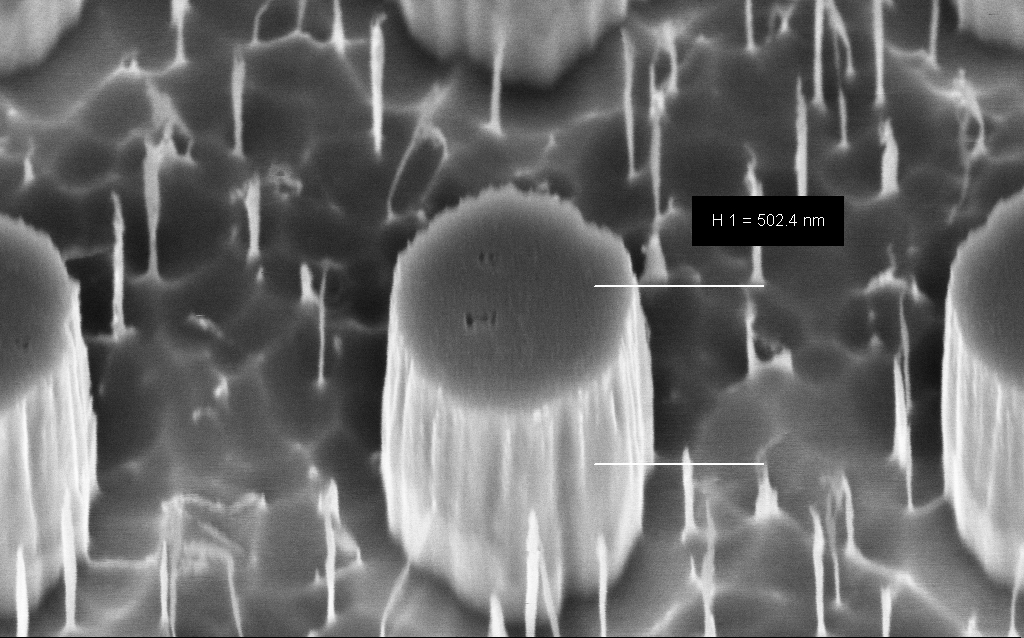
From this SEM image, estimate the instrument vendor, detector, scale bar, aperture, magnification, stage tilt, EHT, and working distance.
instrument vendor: Zeiss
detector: InLens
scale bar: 200 nm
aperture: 30 µm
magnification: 130.1 K X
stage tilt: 45°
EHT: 3 kV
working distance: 5 mm